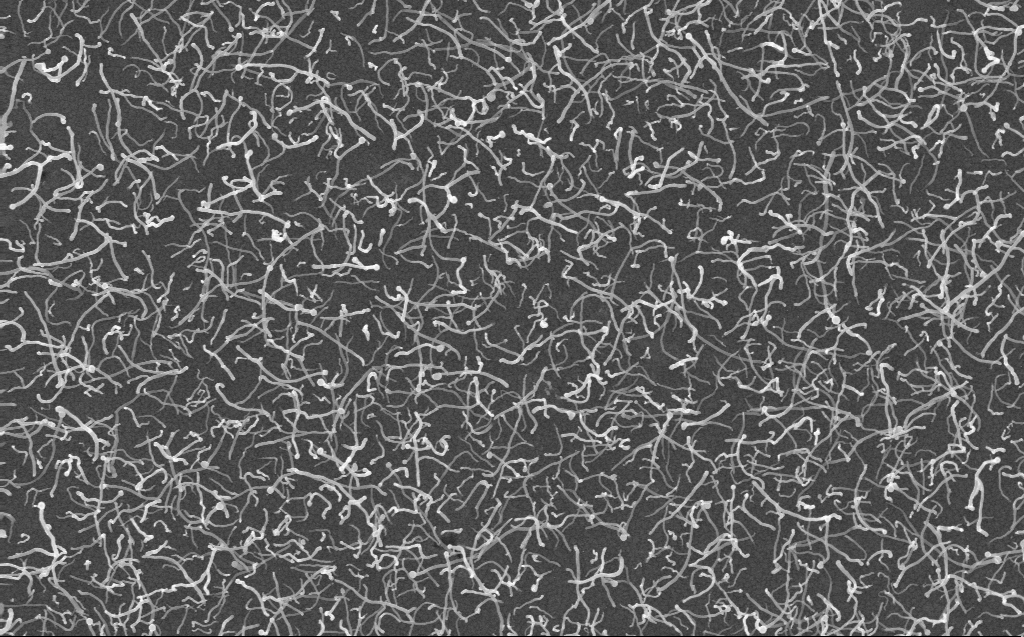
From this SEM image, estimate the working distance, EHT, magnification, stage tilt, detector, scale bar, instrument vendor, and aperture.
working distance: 3 mm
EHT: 10 kV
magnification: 20 K X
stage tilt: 0°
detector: InLens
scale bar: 2000 nm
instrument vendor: Zeiss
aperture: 30 µm